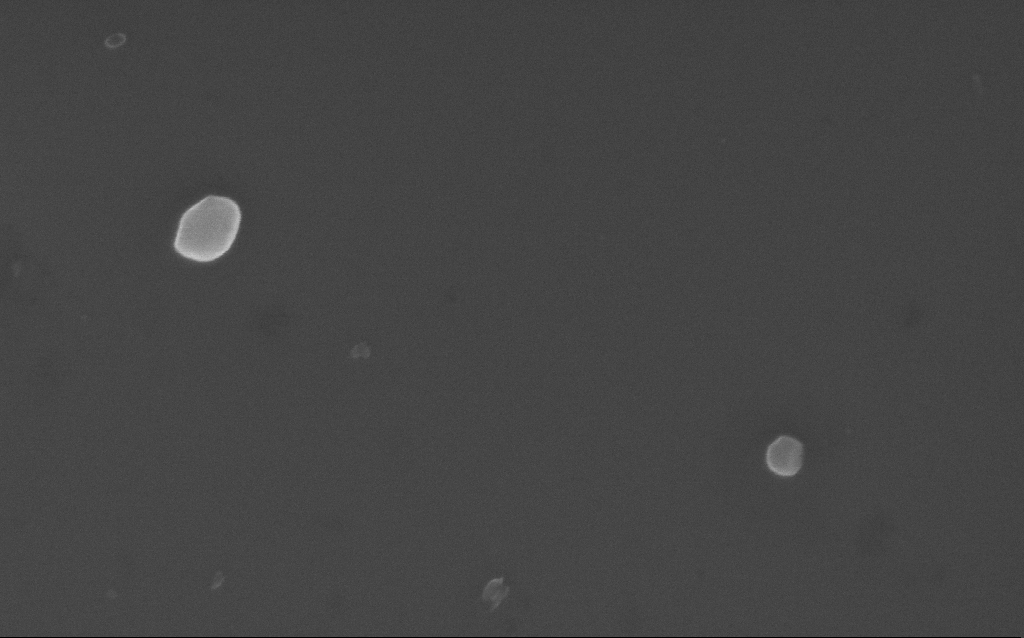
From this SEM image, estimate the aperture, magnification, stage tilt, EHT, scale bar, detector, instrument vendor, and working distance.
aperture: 30 µm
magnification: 125.24 K X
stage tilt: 0°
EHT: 10 kV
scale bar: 200 nm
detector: InLens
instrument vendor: Zeiss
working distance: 3 mm